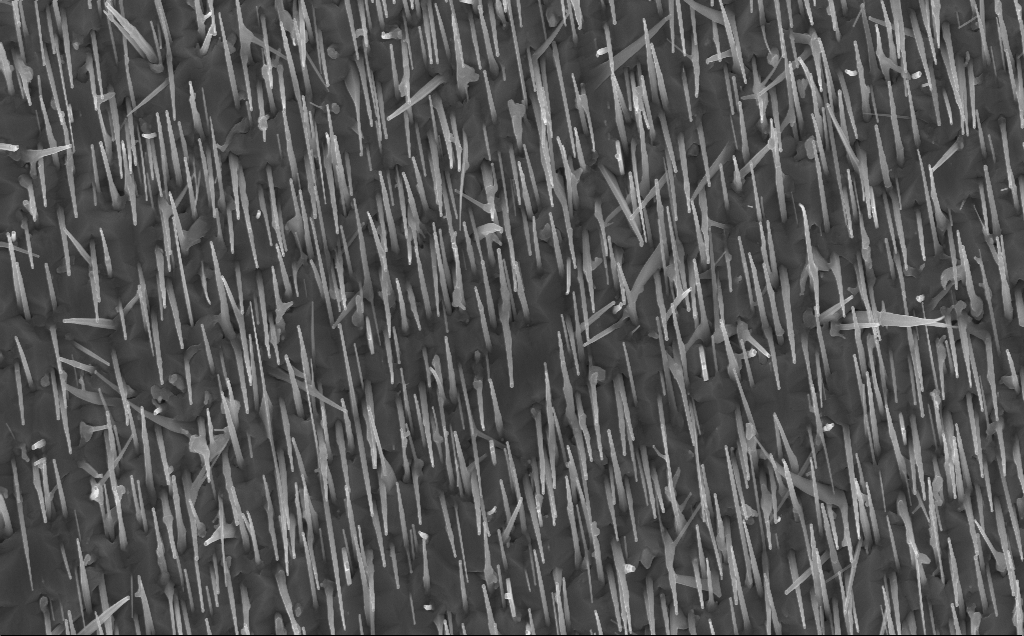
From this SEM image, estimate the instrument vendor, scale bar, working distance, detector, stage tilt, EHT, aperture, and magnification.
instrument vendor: Zeiss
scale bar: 1000 nm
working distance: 7 mm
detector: InLens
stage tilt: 0°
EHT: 10 kV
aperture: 30 µm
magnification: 20 K X